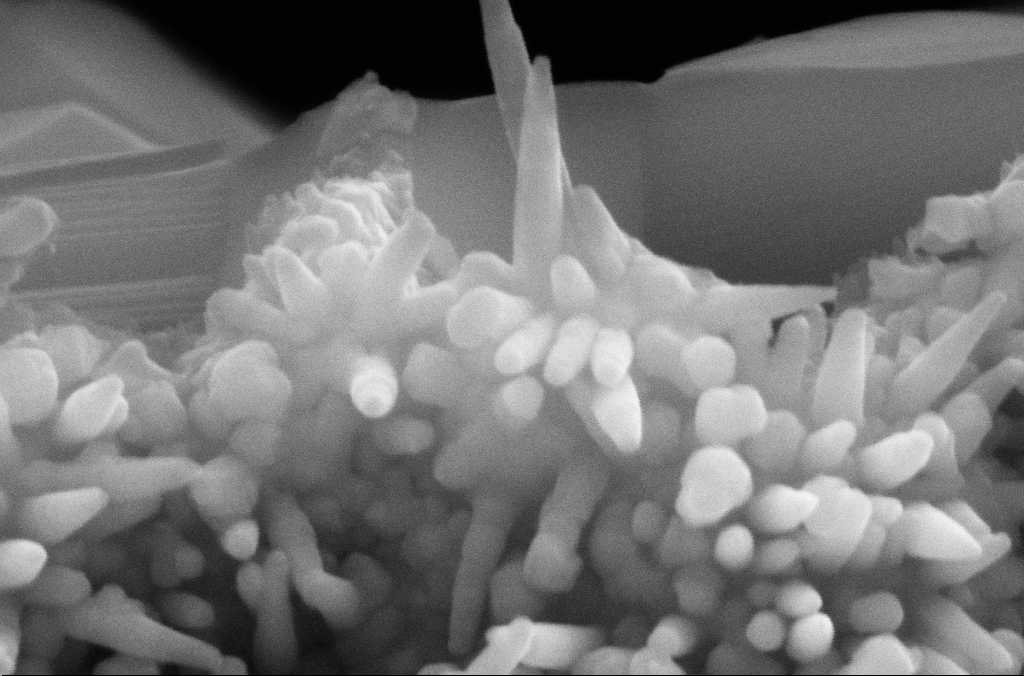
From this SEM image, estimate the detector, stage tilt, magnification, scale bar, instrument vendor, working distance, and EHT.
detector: SE2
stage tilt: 0.1°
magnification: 291.23 K X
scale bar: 100 nm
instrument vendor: Zeiss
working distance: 5.2 mm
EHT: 10 kV